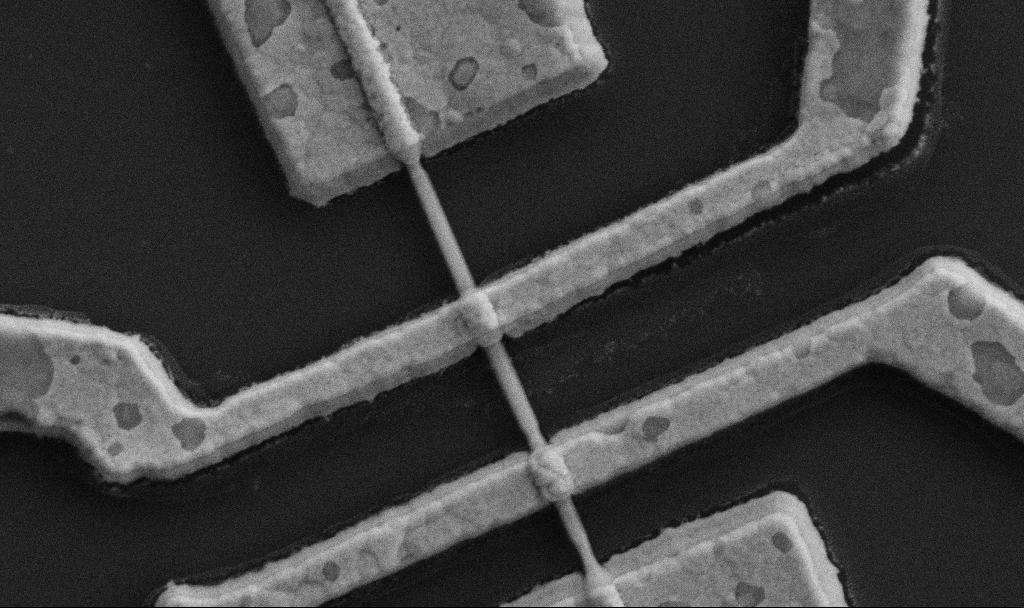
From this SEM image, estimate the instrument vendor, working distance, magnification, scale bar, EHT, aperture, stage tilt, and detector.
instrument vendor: Zeiss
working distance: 9.7 mm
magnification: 60 K X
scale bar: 1000 nm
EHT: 5 kV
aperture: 30 µm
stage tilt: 0°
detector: SE2